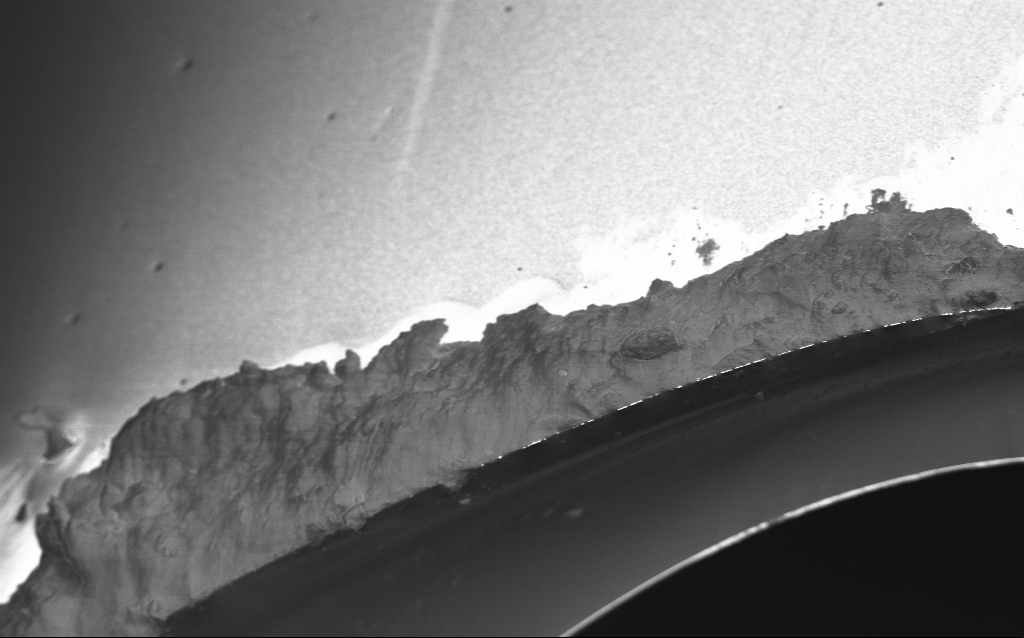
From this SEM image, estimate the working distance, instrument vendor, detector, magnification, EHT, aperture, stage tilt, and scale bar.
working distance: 5 mm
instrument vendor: Zeiss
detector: InLens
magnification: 4.39 K X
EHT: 1 kV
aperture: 30 µm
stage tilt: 45°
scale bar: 10000 nm